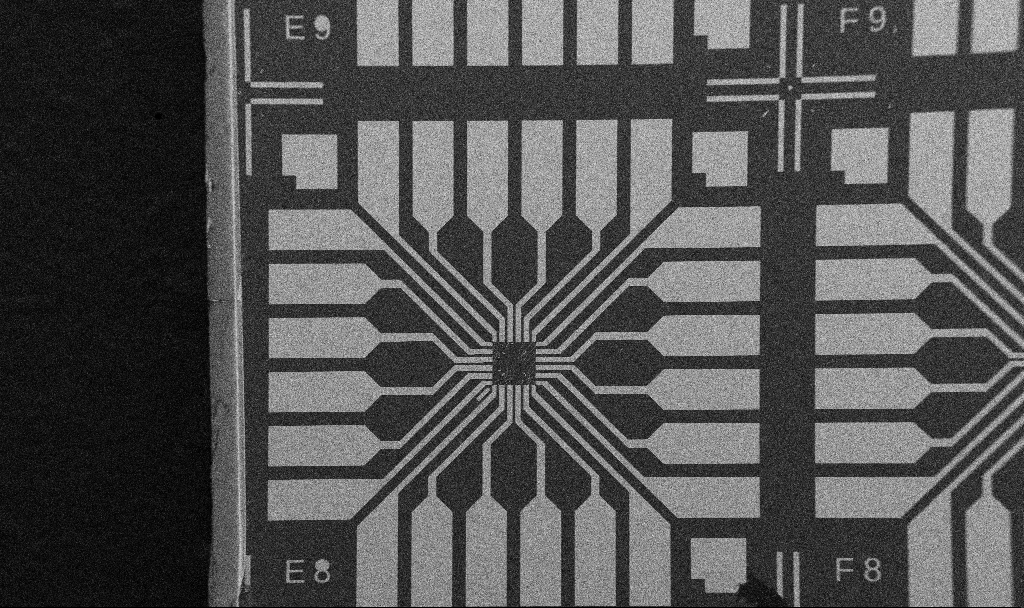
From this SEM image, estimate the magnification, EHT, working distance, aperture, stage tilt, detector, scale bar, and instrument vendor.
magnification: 0.1 K X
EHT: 5 kV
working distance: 10.7 mm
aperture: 30 µm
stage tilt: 0°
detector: SE2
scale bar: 200000 nm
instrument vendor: Zeiss